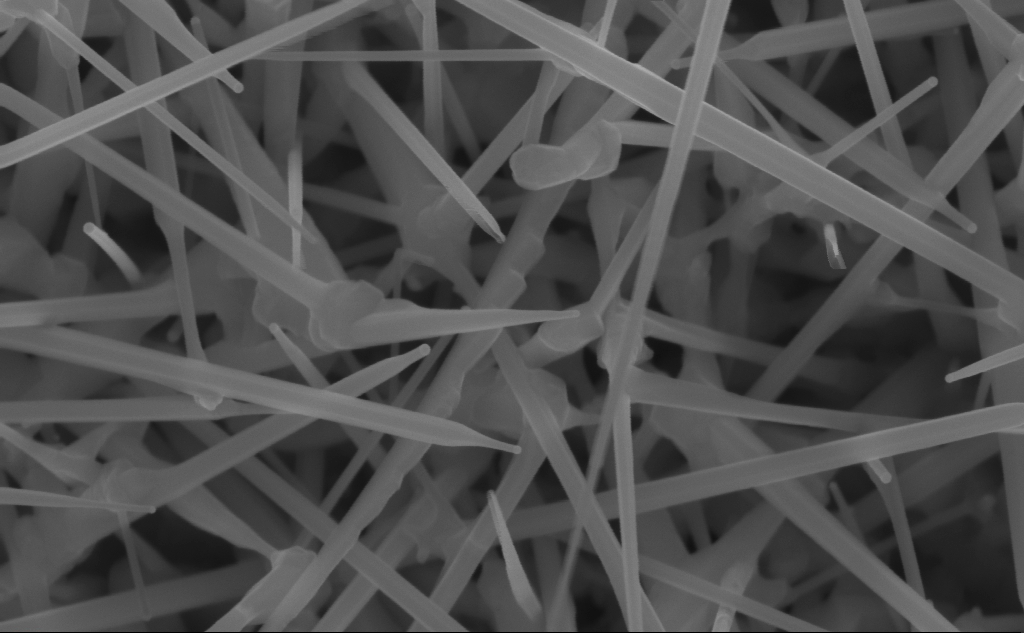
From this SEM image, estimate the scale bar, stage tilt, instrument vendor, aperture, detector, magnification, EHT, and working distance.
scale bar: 200 nm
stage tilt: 0°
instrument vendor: Zeiss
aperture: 30 µm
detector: InLens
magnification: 120.77 K X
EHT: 10 kV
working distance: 7 mm